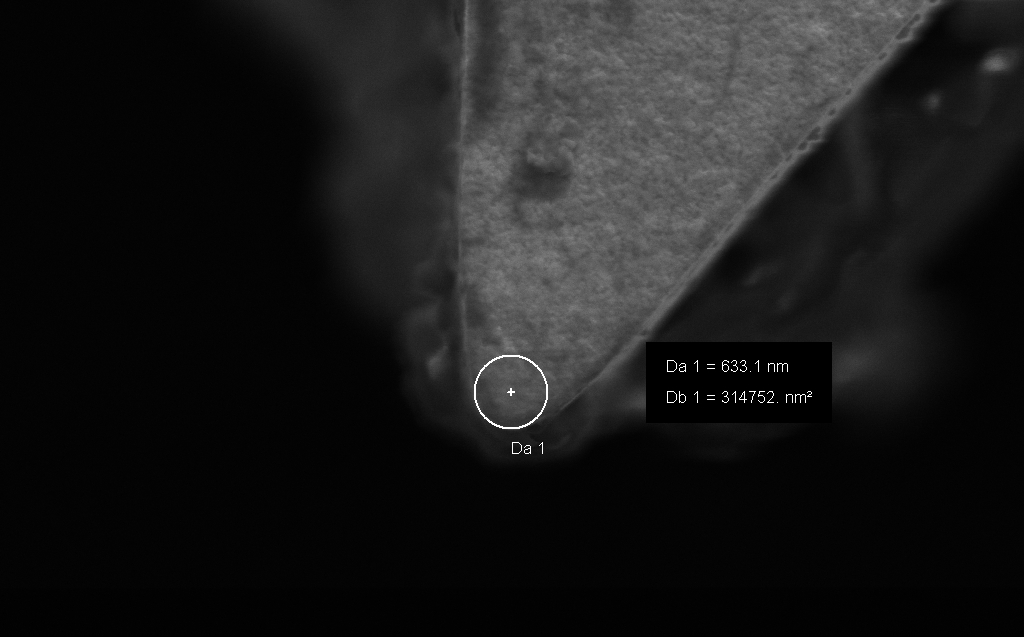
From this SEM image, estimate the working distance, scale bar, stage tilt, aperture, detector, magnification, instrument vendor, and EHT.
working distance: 8 mm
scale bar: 1000 nm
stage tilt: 0°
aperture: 30 µm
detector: InLens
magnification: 42.92 K X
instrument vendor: Zeiss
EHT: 3 kV